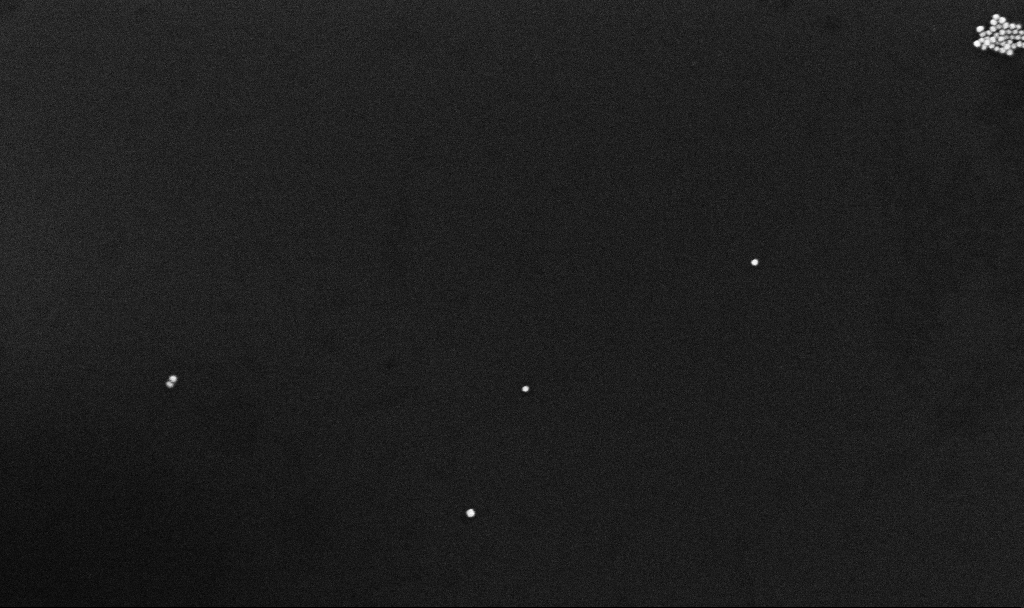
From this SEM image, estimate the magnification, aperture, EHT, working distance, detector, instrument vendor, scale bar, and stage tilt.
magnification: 105.84 K X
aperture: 30 µm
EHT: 10 kV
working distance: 3.3 mm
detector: InLens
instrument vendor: Zeiss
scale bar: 200 nm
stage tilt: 0°